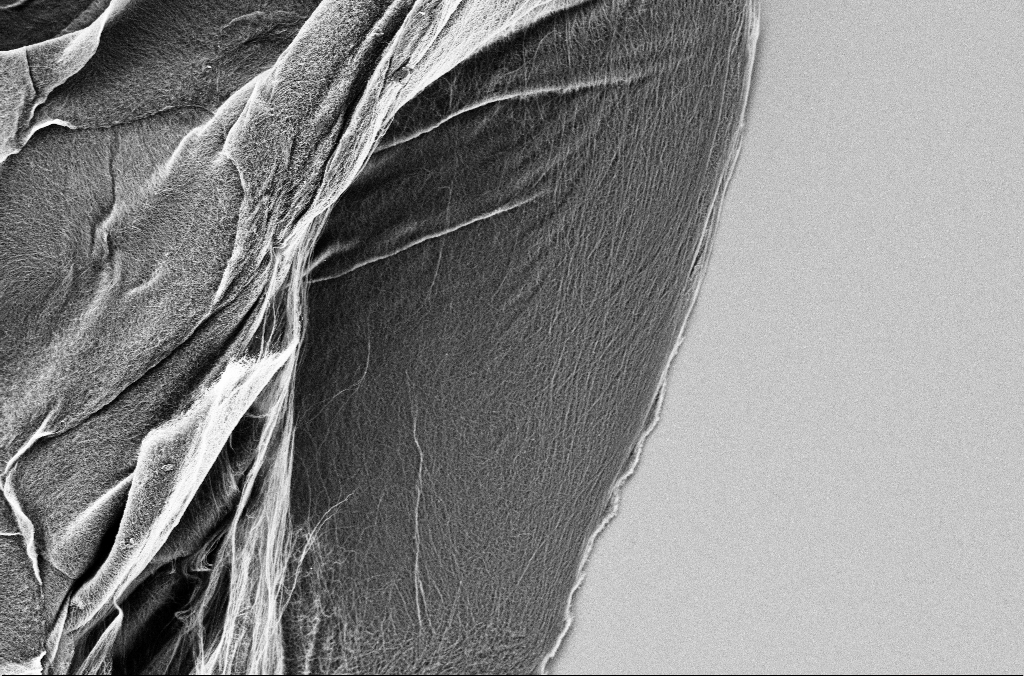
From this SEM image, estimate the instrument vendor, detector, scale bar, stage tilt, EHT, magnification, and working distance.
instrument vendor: Zeiss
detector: SE2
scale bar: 10000 nm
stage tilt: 0°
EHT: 1.8 kV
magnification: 5 K X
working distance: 5.7 mm